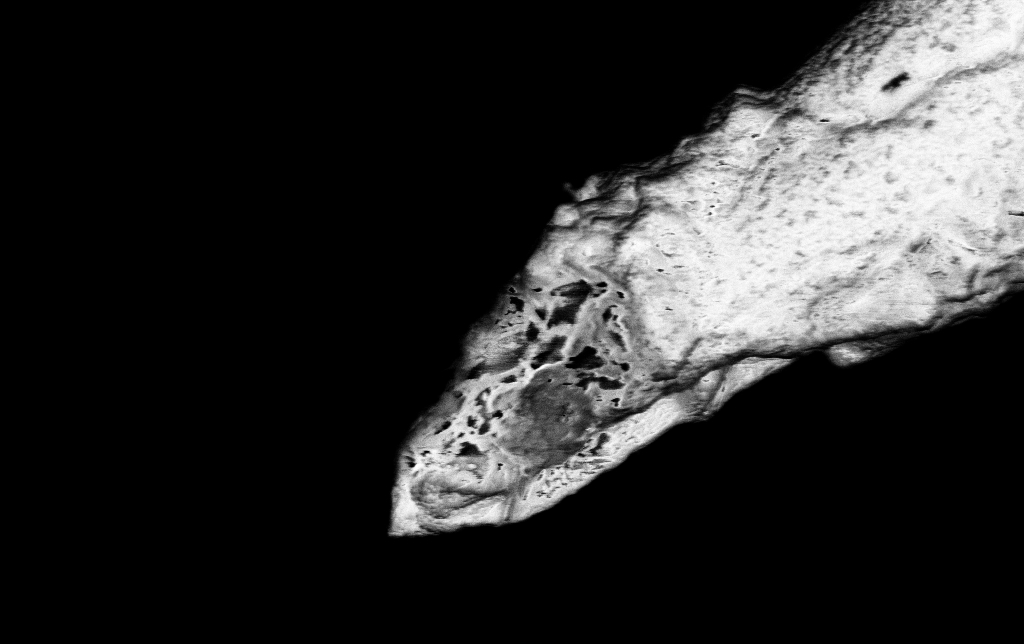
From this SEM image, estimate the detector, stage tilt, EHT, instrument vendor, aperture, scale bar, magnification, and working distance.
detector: InLens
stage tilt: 0°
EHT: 3 kV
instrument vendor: Zeiss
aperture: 30 µm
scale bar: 1000 nm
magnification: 25 K X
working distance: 7.5 mm